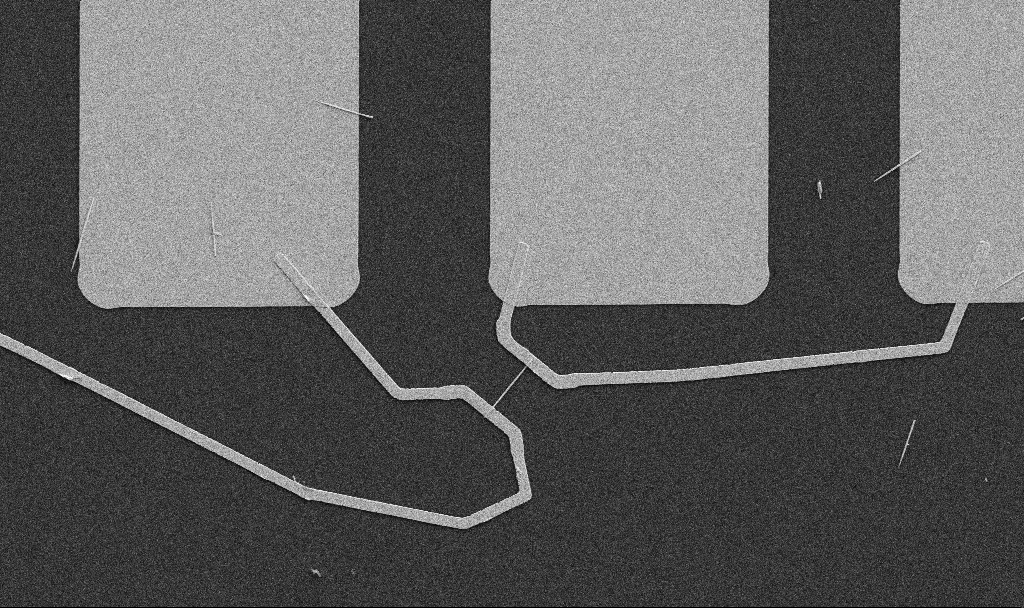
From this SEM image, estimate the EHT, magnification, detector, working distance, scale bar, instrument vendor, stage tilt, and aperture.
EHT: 5 kV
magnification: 5 K X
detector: SE2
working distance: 10.7 mm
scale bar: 10000 nm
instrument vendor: Zeiss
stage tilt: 0°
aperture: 30 µm